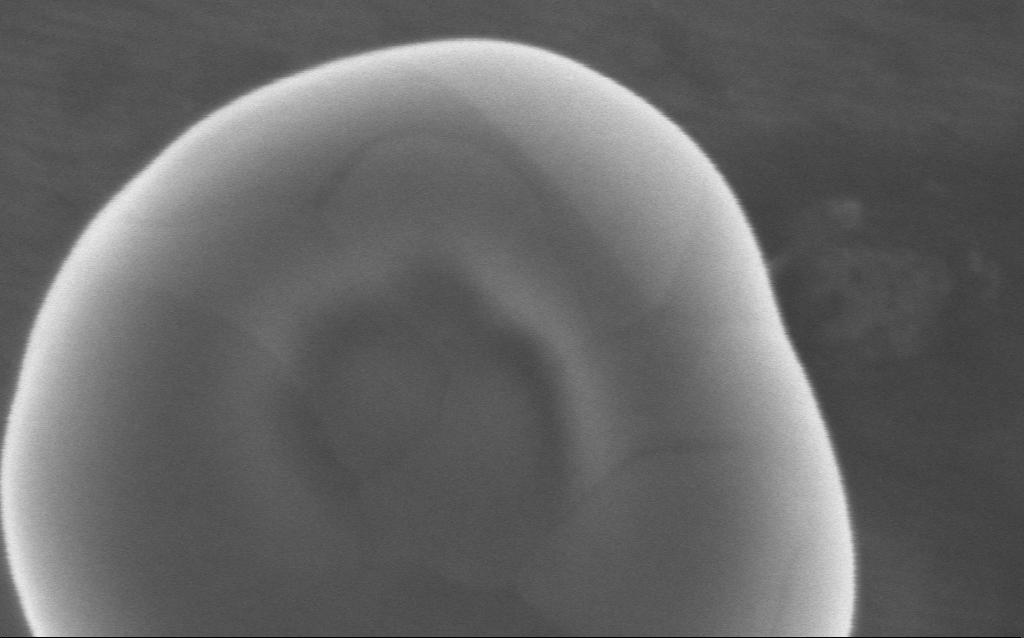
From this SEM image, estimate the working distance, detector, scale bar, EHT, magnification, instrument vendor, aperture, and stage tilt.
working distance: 4 mm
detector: InLens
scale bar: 100 nm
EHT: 5 kV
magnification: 451.31 K X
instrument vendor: Zeiss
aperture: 30 µm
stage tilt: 0°